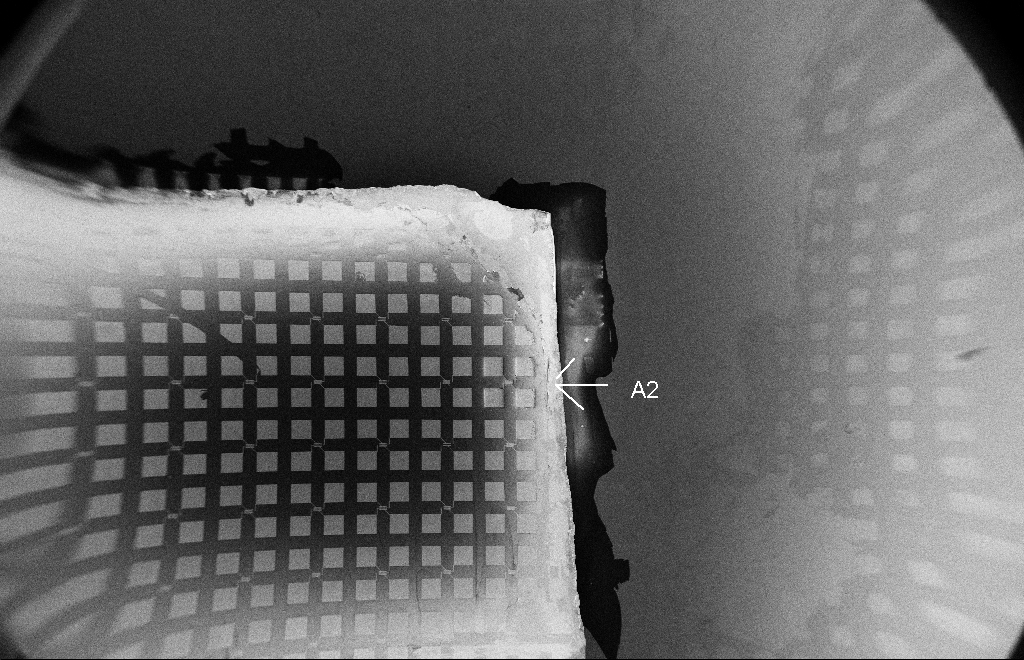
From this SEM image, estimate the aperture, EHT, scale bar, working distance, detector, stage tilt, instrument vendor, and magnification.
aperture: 10 µm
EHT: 5 kV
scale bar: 200000 nm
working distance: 16 mm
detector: SE2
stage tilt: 0°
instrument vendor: Zeiss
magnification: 0.048 K X